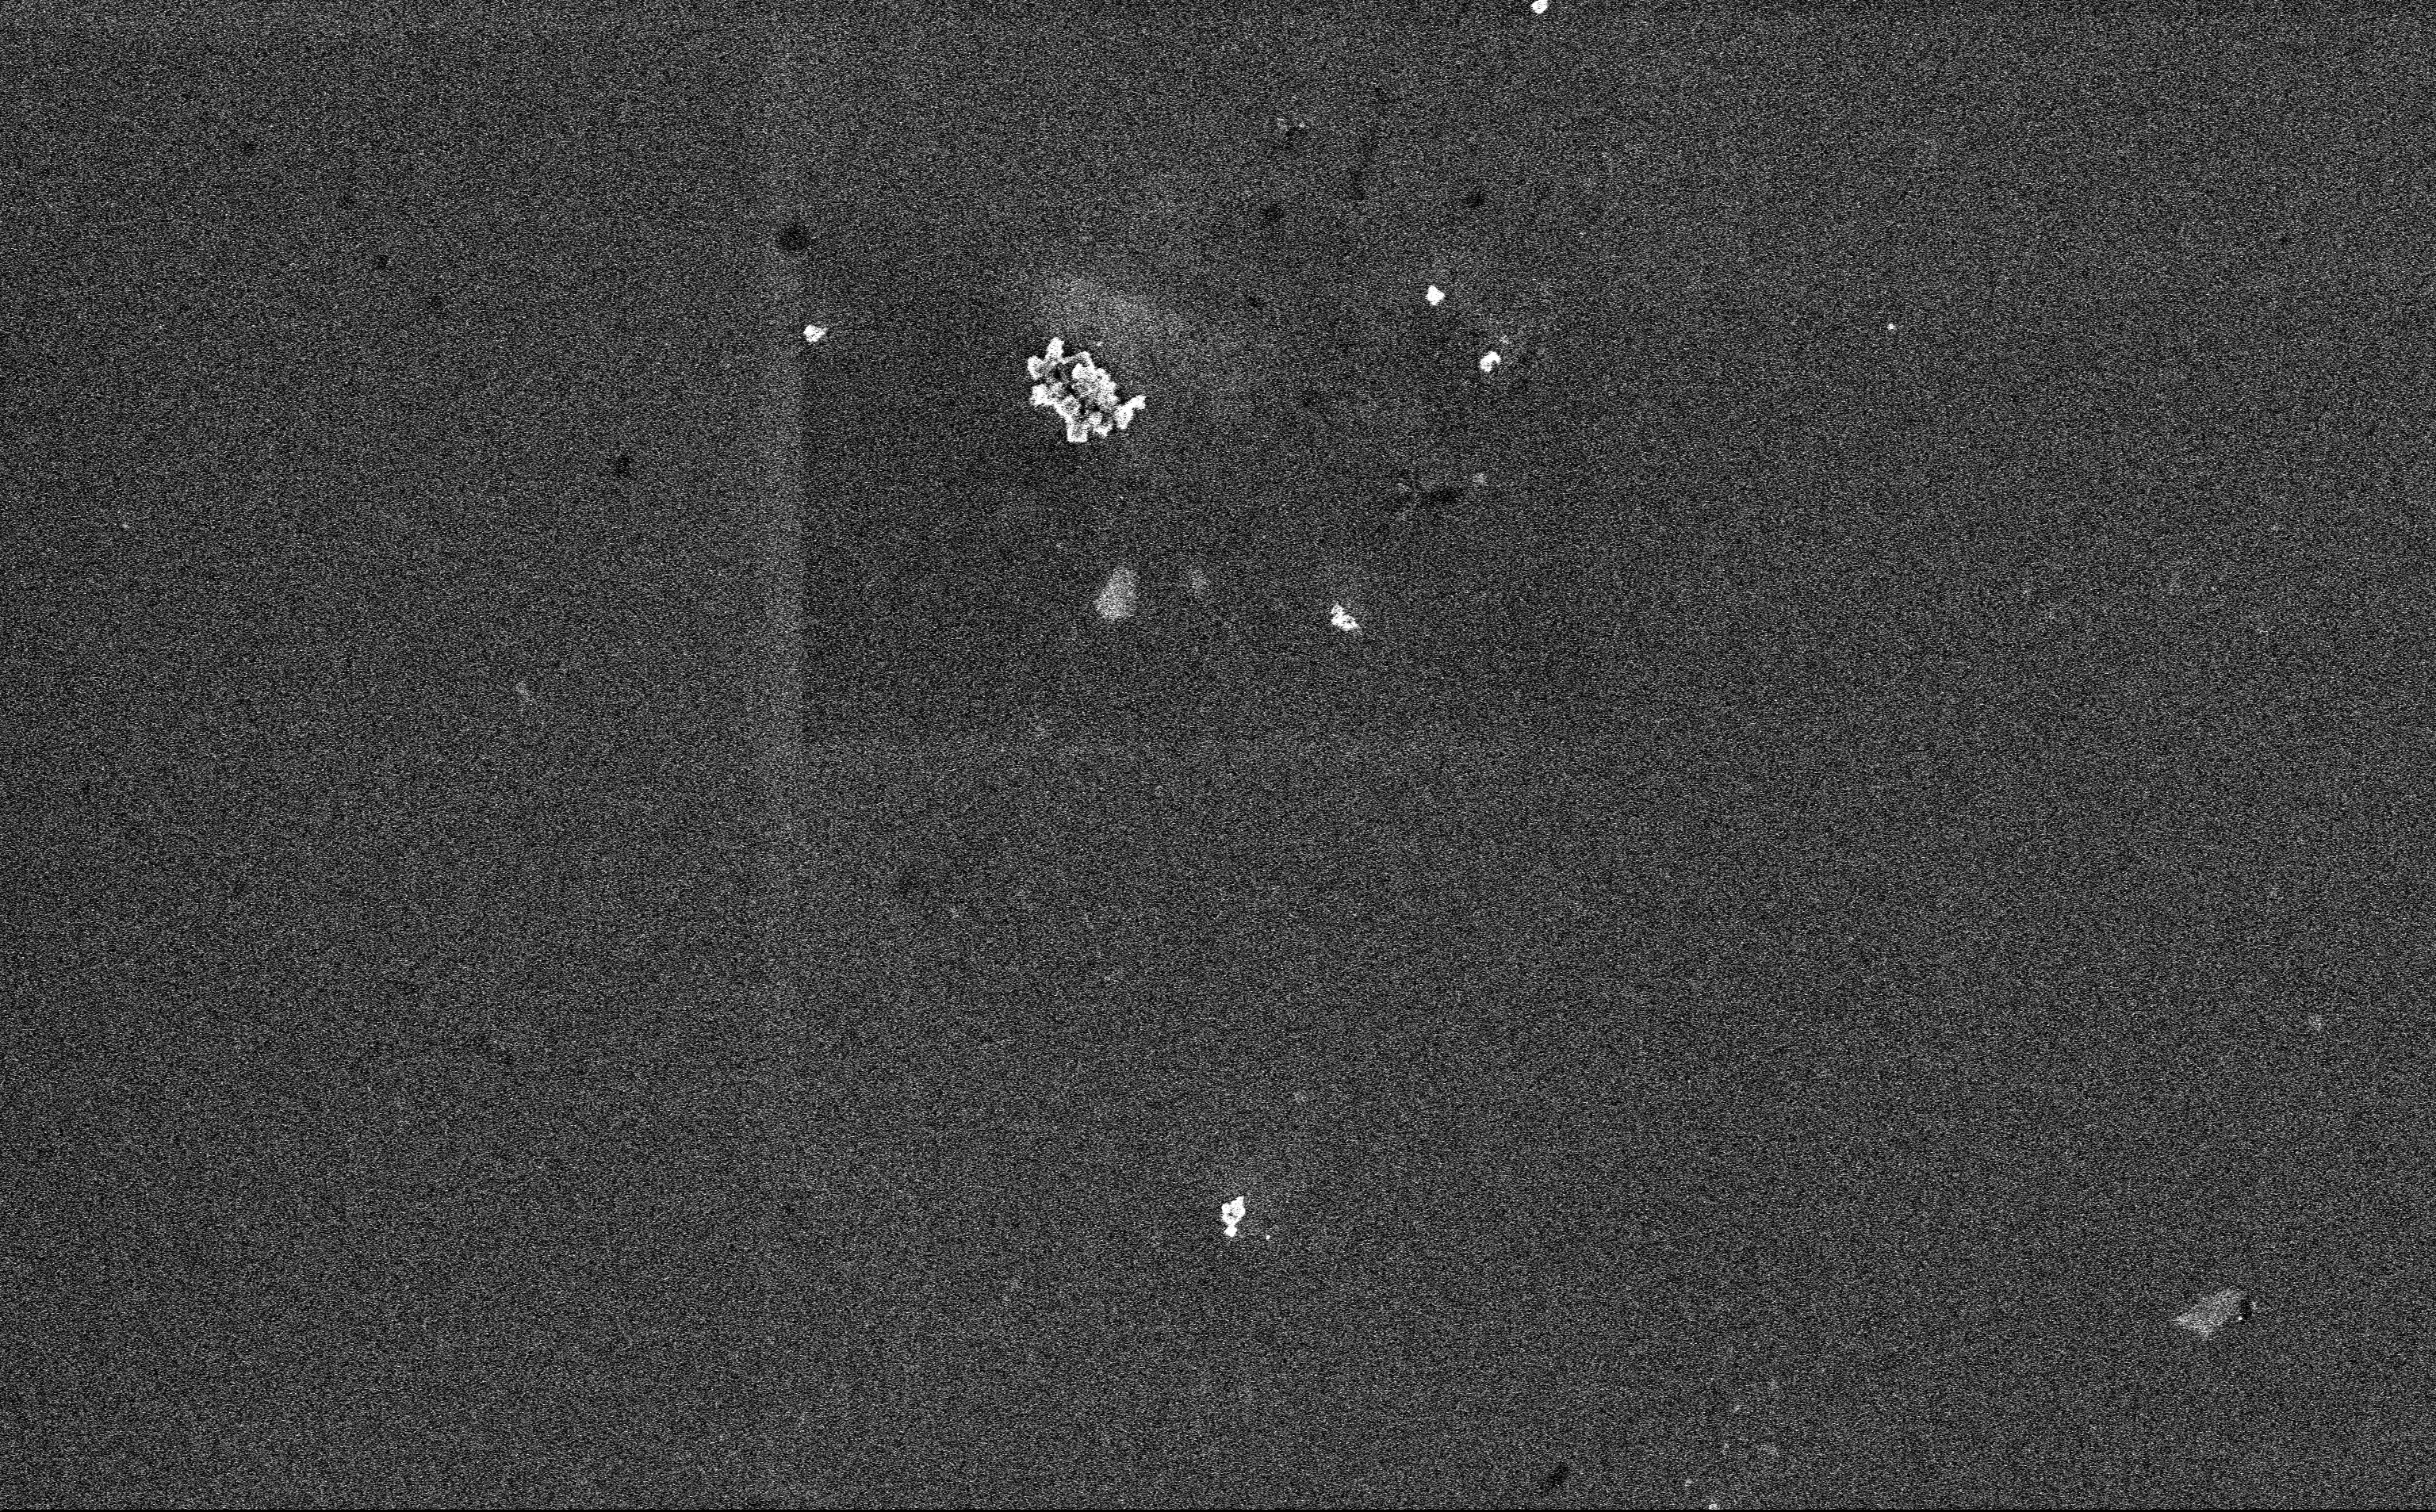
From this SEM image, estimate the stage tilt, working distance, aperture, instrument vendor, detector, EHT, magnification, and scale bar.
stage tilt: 0°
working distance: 3 mm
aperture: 30 µm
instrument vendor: Zeiss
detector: InLens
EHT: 3 kV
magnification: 12.85 K X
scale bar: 2000 nm